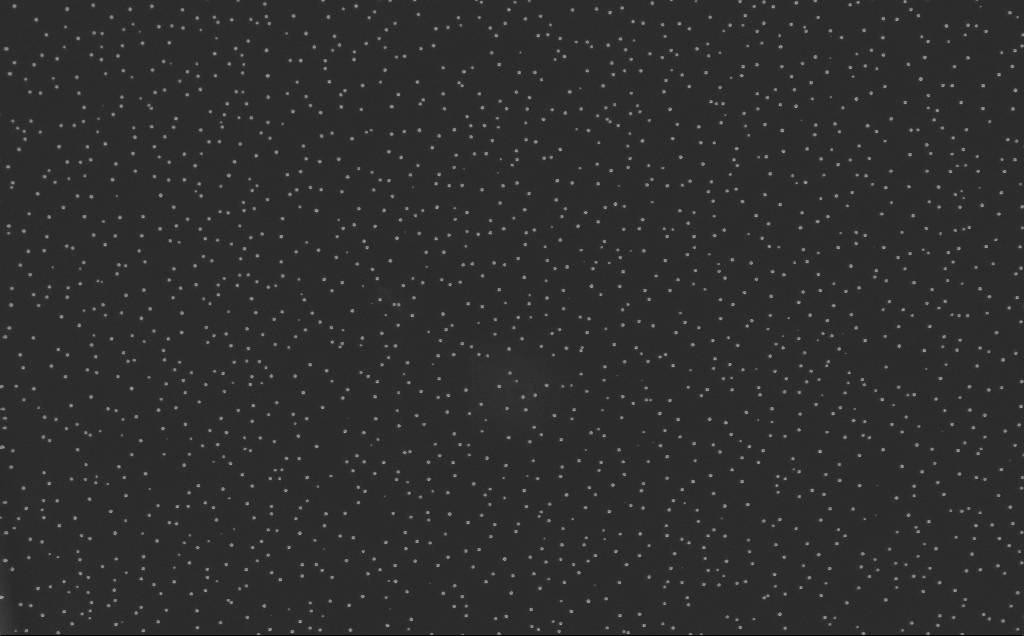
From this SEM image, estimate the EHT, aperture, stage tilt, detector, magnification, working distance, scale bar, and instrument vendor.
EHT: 10 kV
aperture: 30 µm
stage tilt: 0°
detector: InLens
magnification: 20 K X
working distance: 8 mm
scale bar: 1000 nm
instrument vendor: Zeiss